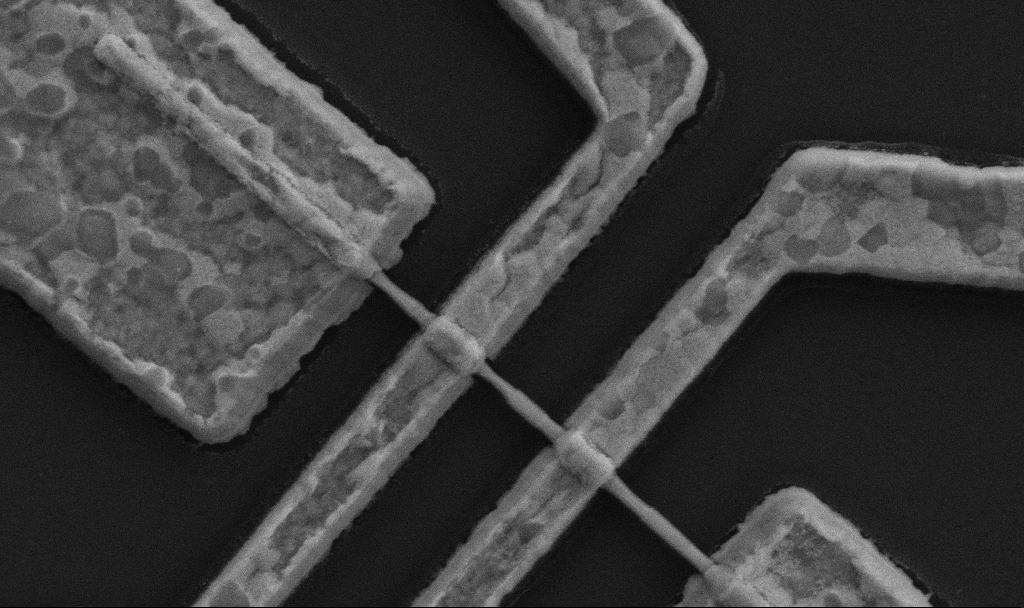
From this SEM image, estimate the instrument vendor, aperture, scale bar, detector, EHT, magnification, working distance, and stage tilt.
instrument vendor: Zeiss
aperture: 30 µm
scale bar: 1000 nm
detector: SE2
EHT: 5 kV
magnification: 60 K X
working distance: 10.7 mm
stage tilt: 0°